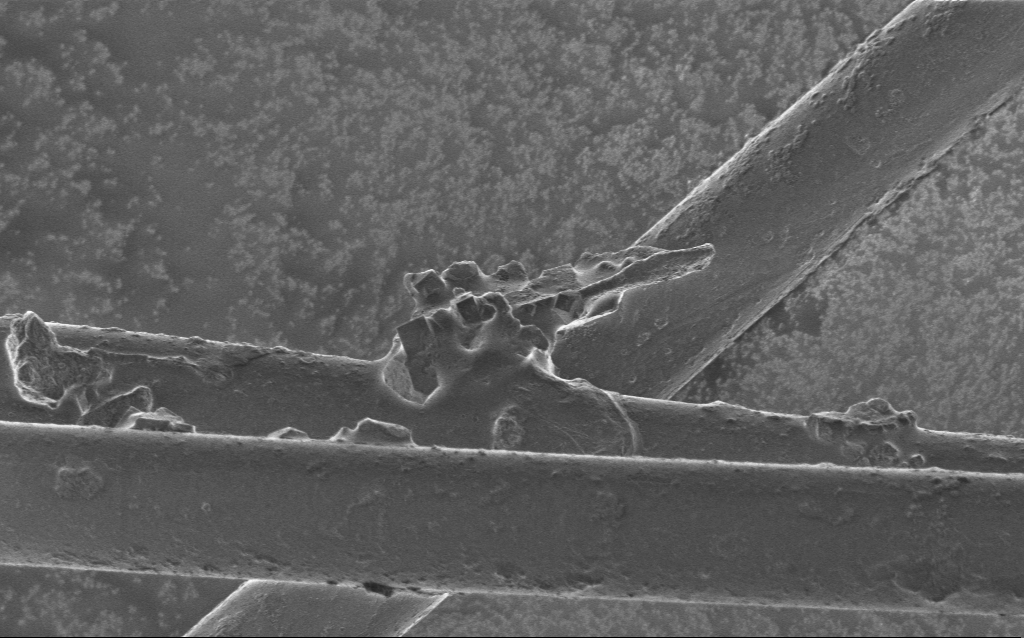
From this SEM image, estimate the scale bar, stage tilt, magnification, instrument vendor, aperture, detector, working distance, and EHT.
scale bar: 10000 nm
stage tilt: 0°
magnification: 2.28 K X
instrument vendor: Zeiss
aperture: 30 µm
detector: InLens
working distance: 5 mm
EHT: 1 kV